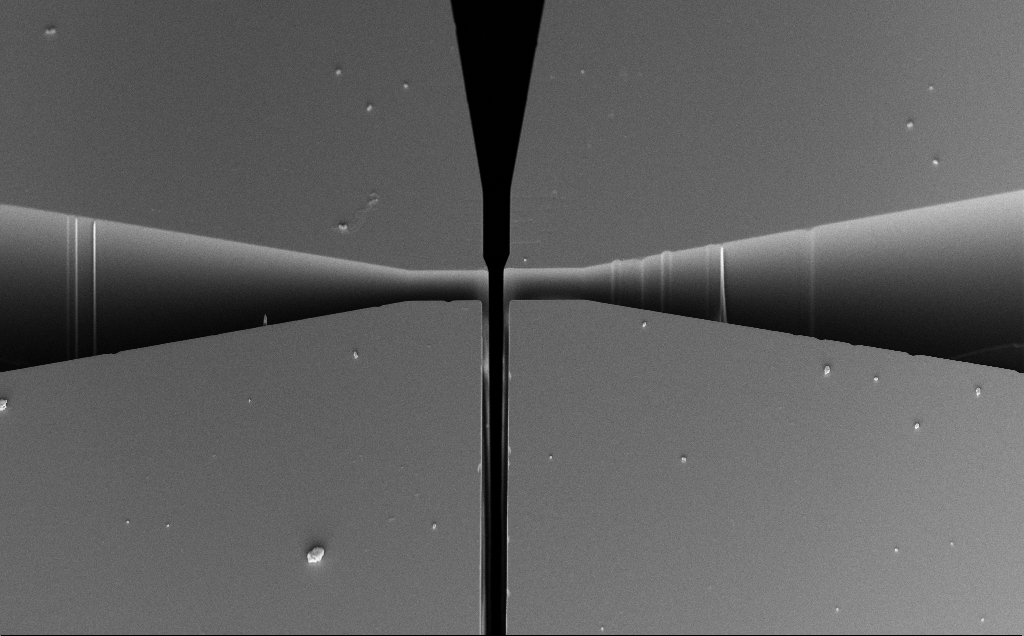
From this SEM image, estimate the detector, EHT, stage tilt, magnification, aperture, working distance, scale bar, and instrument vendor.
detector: InLens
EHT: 5 kV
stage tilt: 44.8°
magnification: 0.82 K X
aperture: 30 µm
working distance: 7 mm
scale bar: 20000 nm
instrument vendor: Zeiss